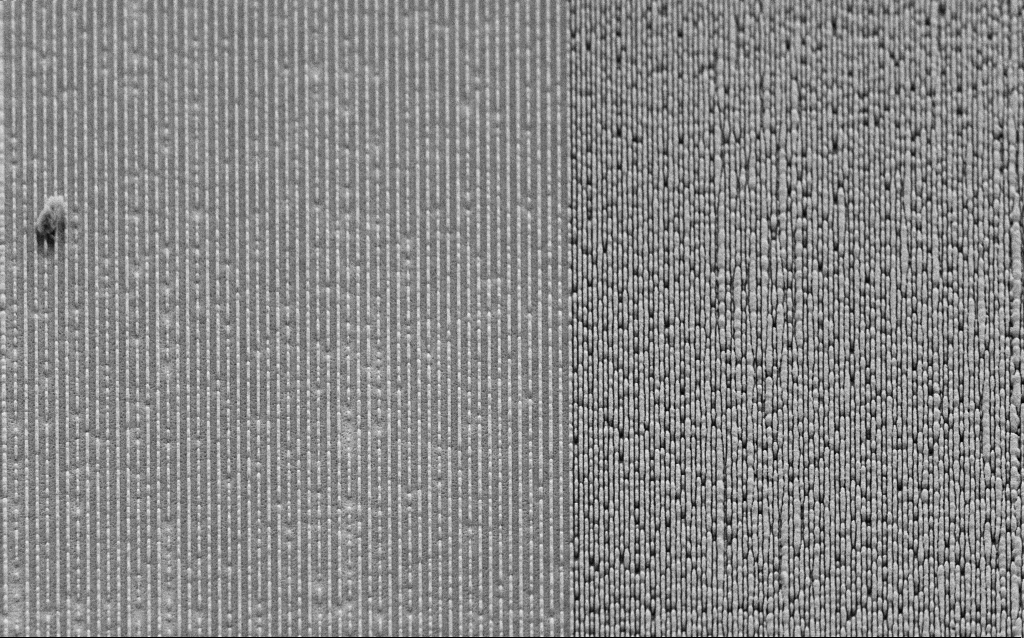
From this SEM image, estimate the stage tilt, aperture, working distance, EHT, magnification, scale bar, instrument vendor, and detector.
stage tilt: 45°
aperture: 30 µm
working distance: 6.6 mm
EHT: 3 kV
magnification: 18.51 K X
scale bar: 1000 nm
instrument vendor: Zeiss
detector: SE2